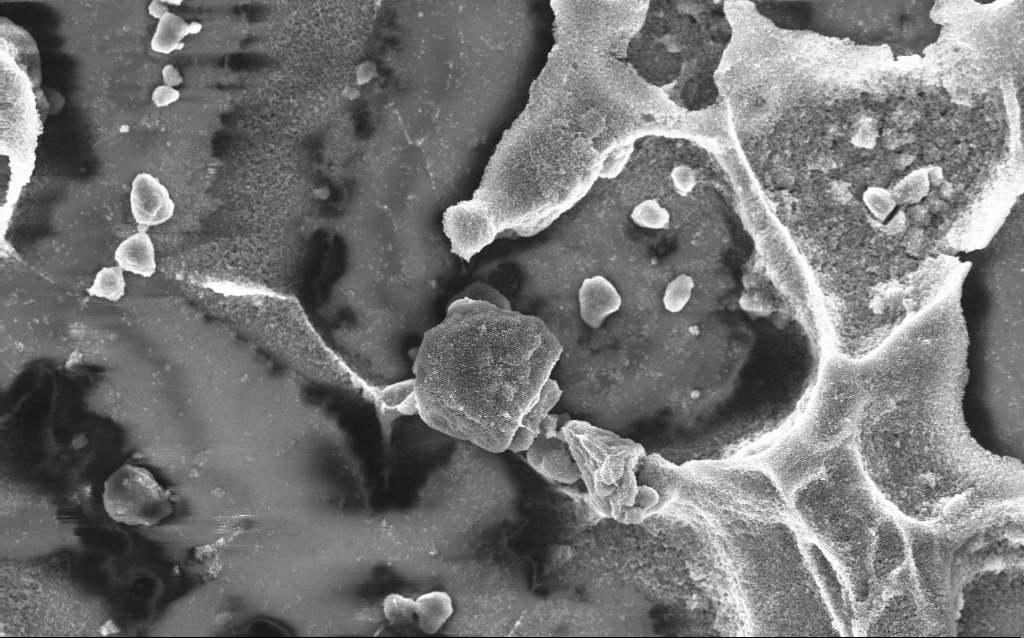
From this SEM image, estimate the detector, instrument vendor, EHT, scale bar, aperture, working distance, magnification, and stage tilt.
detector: InLens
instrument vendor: Zeiss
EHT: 10 kV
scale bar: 10000 nm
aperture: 30 µm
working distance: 2.8 mm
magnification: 5.83 K X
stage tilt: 0°